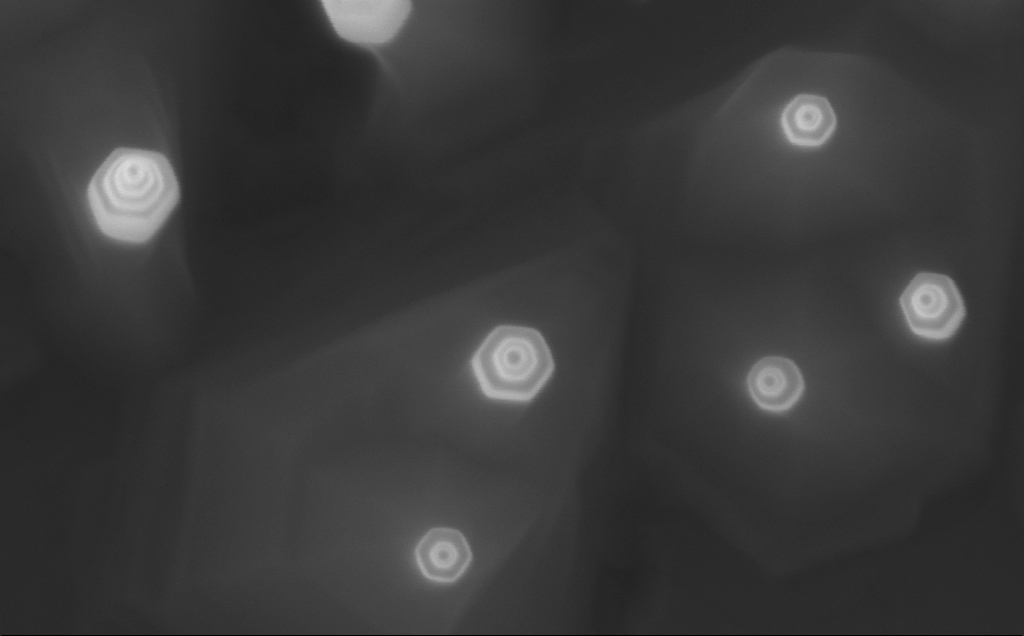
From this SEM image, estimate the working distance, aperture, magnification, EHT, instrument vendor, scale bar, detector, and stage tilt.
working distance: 5 mm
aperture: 30 µm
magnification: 300 K X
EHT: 10 kV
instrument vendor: Zeiss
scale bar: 100 nm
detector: InLens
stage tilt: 0°